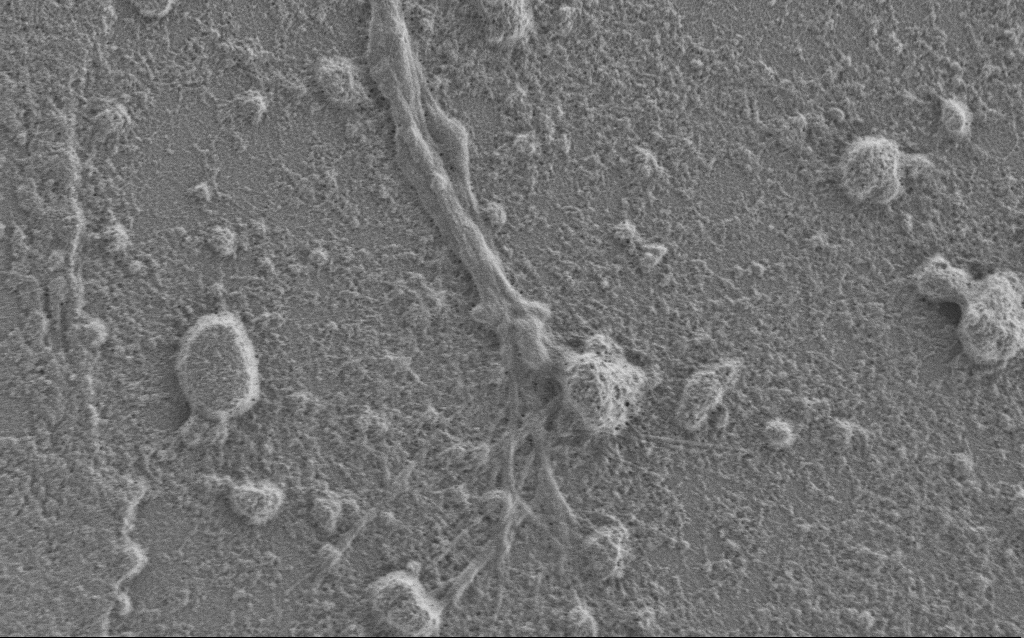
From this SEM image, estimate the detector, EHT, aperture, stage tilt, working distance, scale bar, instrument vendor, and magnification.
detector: SE2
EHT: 1 kV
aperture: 30 µm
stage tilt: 0°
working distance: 6 mm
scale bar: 2000 nm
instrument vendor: Zeiss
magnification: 7.5 K X